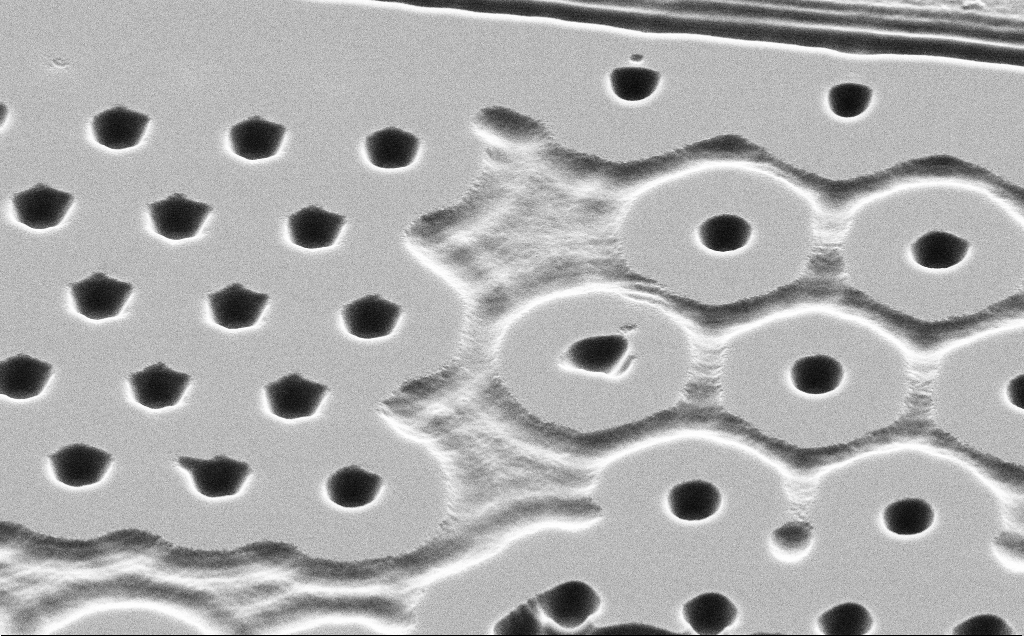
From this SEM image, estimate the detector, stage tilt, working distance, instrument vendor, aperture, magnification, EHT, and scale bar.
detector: SE2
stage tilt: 45°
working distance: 11 mm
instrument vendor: Zeiss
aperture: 30 µm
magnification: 7.86 K X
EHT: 5 kV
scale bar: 2000 nm